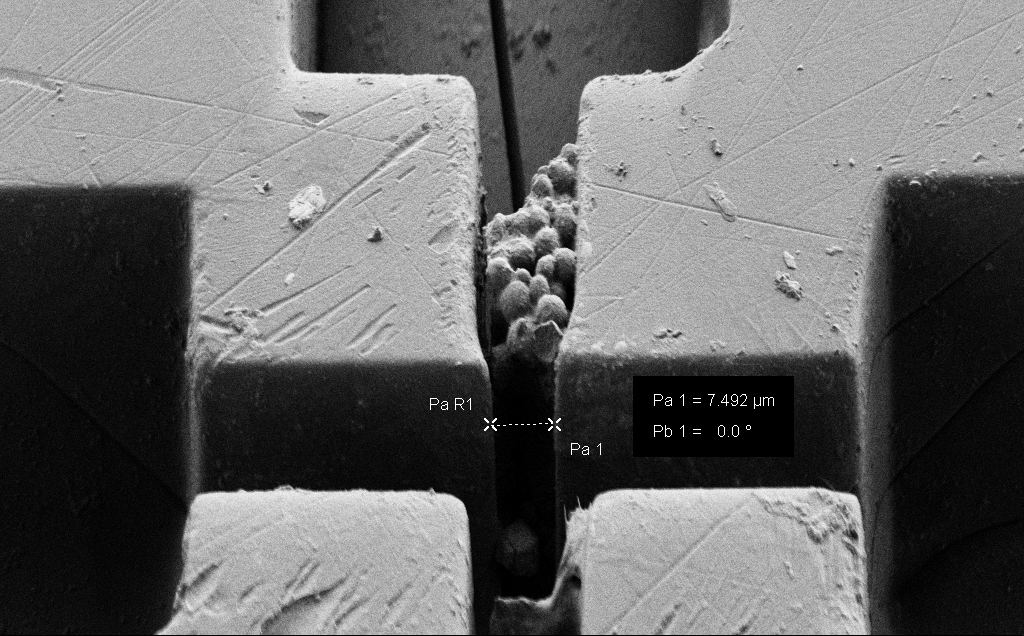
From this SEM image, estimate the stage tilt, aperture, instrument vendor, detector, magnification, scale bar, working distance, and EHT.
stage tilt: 45°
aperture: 30 µm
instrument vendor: Zeiss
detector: SE2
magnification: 3.14 K X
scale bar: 20000 nm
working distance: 5 mm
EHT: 1 kV